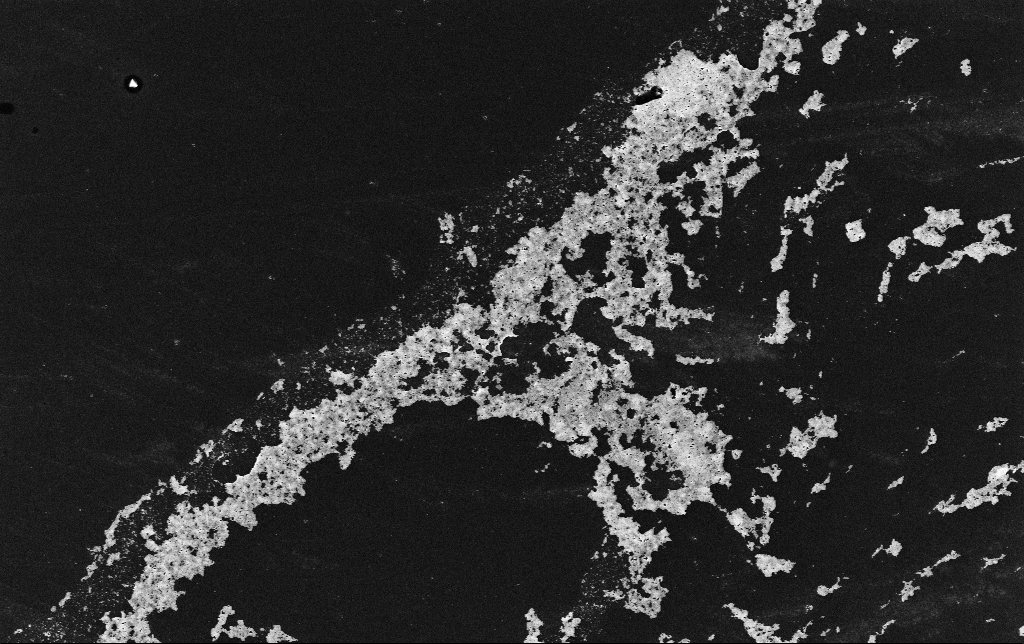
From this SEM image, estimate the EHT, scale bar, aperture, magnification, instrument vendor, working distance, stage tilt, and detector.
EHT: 10 kV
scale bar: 10000 nm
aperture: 30 µm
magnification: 2.72 K X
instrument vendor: Zeiss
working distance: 3.4 mm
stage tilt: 0°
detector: InLens